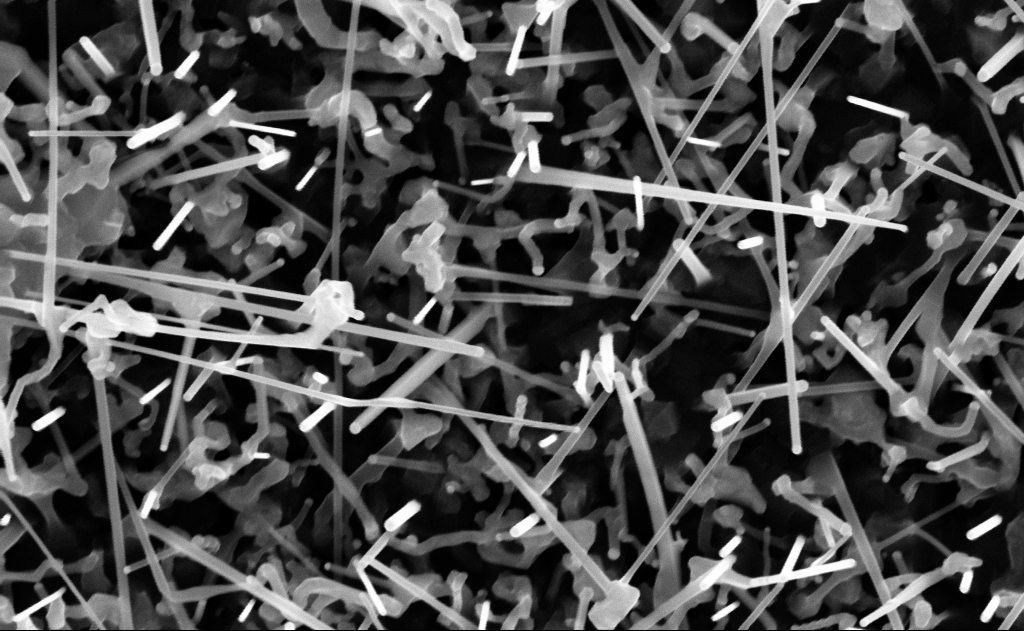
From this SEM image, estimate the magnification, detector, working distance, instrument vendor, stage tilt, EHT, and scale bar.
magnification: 80 K X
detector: InLens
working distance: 10 mm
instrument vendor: Zeiss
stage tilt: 0°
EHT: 10 kV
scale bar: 200 nm